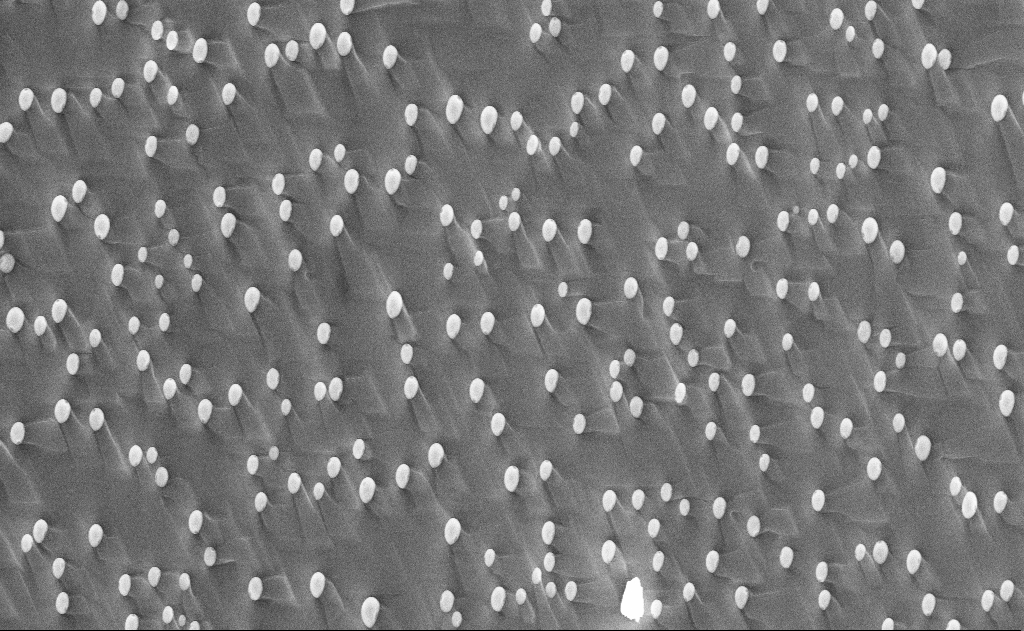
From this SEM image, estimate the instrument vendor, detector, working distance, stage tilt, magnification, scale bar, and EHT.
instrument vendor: Zeiss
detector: InLens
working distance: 12 mm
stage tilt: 0°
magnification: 10 K X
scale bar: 2000 nm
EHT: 10 kV